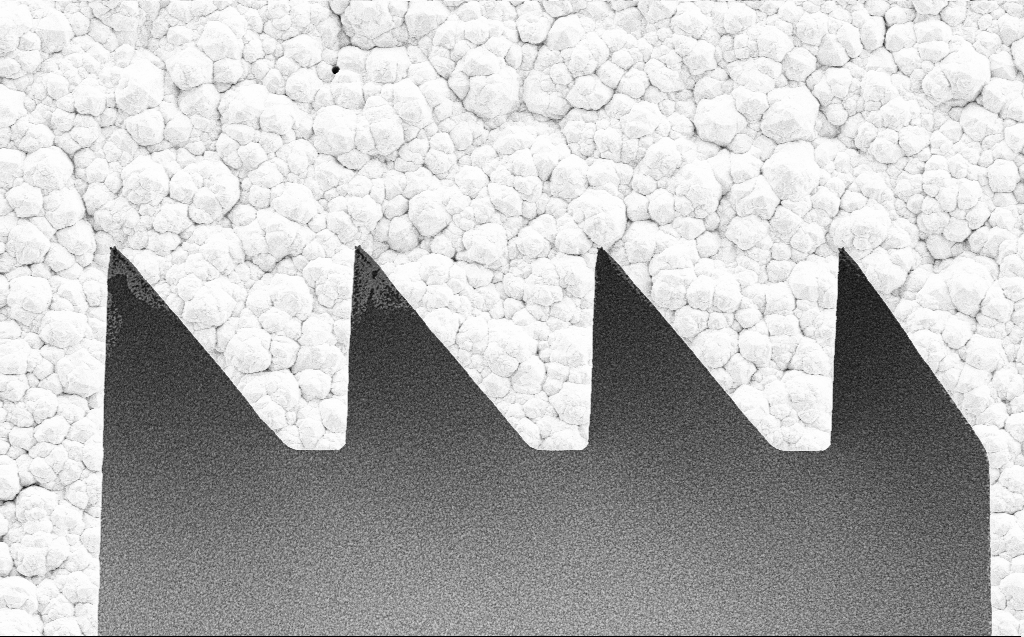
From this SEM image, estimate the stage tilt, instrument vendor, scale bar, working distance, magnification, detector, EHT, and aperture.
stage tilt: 0°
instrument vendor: Zeiss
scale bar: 2000 nm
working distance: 6 mm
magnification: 7.85 K X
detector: SE2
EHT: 5 kV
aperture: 30 µm